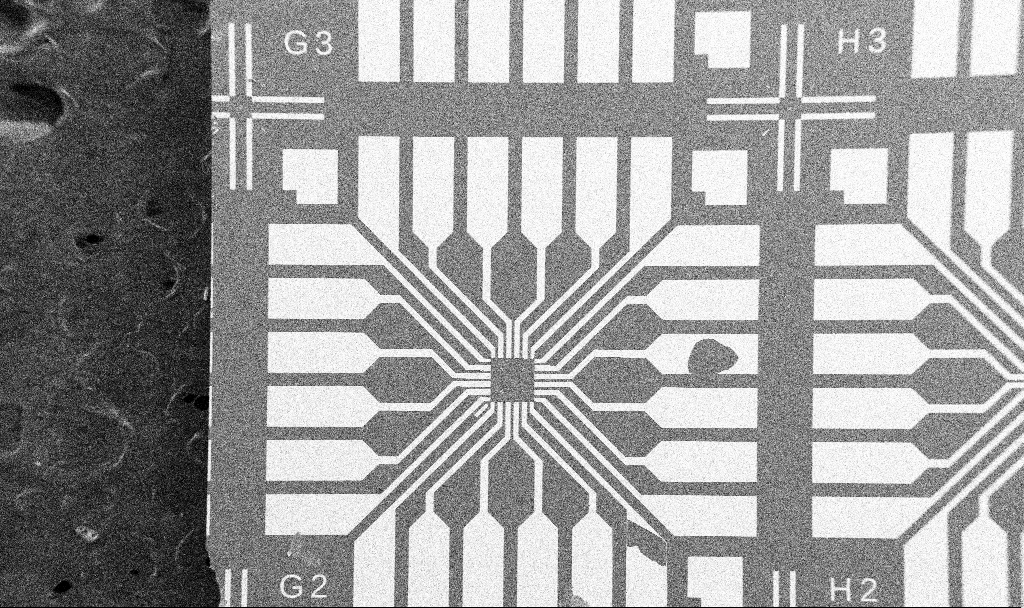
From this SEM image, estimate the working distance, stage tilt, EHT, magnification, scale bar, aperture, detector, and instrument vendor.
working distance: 10.7 mm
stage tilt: -0°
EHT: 5 kV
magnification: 0.1 K X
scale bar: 200000 nm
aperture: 30 µm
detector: SE2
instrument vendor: Zeiss